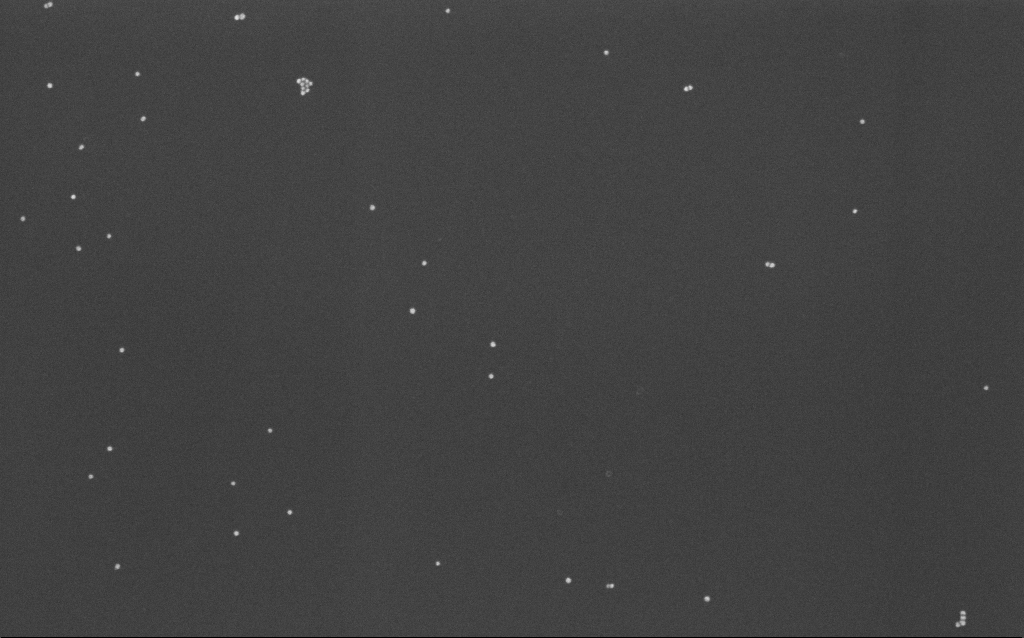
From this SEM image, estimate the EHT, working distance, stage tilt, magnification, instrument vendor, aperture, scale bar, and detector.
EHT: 10 kV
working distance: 7 mm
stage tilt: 0°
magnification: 100 K X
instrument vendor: Zeiss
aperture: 30 µm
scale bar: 200 nm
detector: InLens